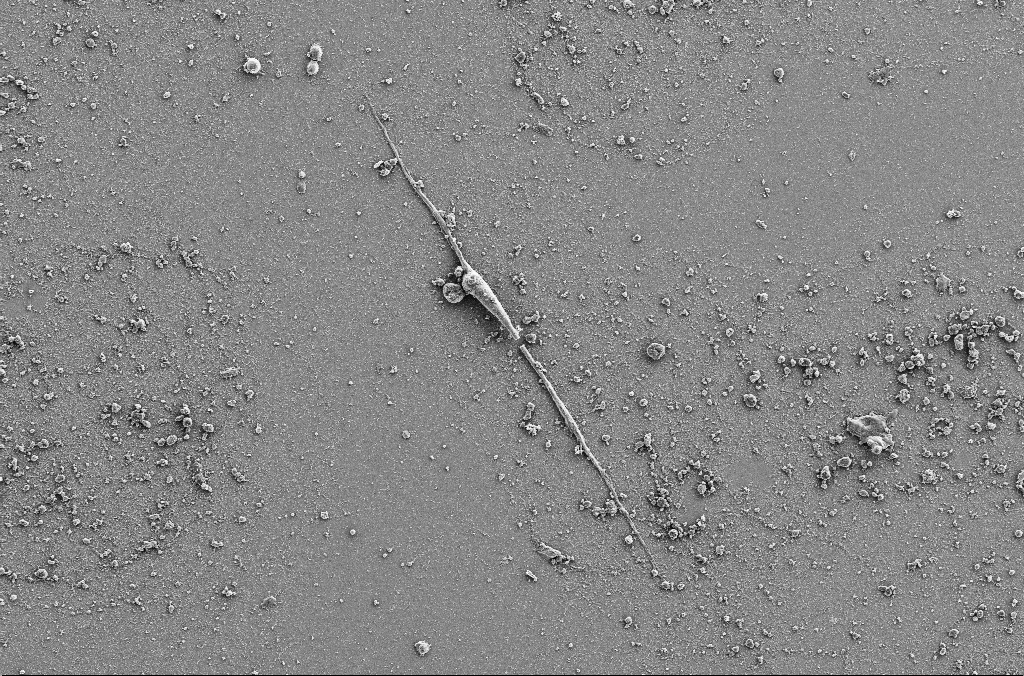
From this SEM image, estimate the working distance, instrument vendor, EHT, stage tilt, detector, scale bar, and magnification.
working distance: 4 mm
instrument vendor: Zeiss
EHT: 5 kV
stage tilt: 0°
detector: SE2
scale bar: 20000 nm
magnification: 1 K X